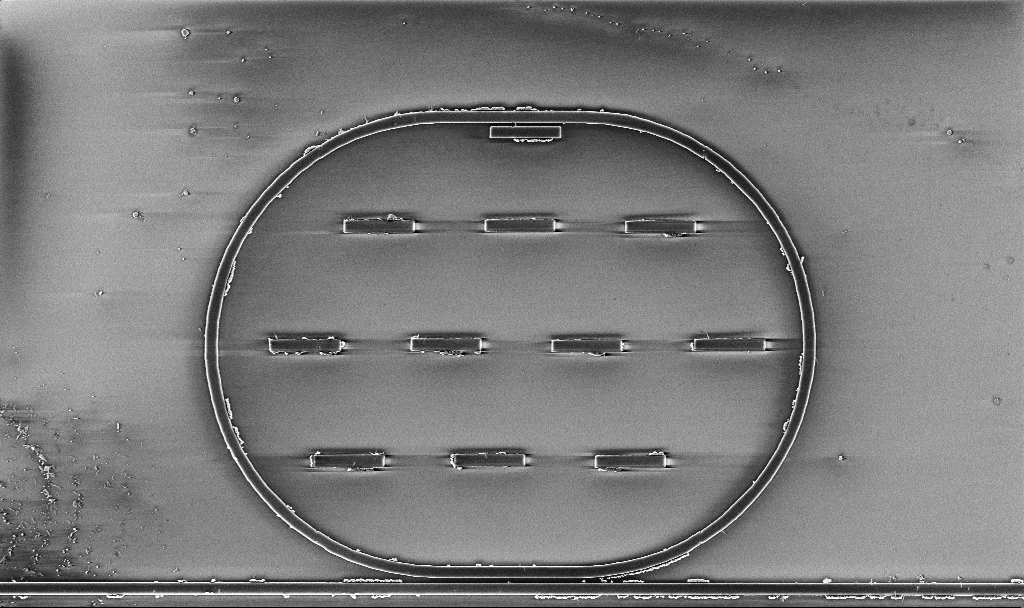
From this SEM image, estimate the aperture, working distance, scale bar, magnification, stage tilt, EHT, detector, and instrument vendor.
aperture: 30 µm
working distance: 3 mm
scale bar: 2000 nm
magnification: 8.71 K X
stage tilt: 0°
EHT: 3 kV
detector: InLens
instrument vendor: Zeiss